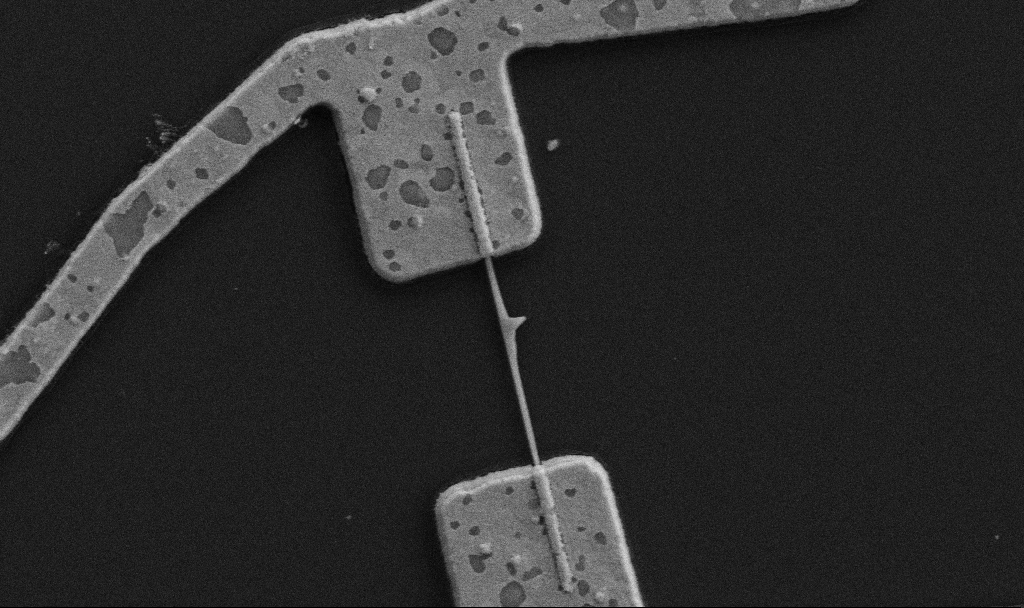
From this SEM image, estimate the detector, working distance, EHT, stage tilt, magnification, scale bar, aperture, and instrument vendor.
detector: SE2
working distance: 8.7 mm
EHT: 5 kV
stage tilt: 0°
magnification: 30 K X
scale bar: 1000 nm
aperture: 30 µm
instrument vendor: Zeiss